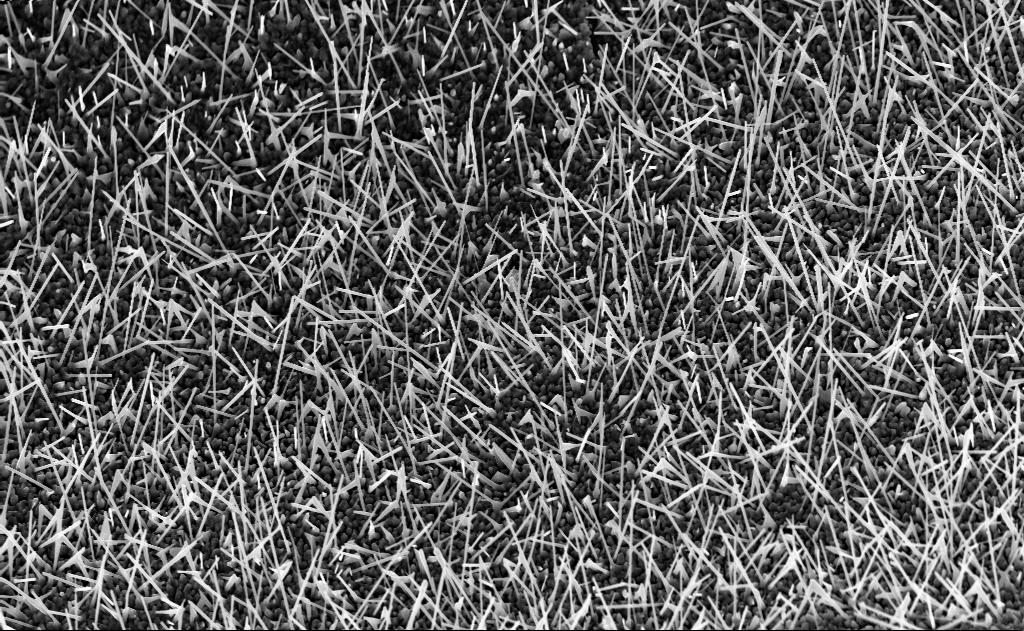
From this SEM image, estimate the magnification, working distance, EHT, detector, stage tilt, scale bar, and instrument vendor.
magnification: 10 K X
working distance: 6 mm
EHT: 10 kV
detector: InLens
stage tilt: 0°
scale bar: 2000 nm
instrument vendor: Zeiss